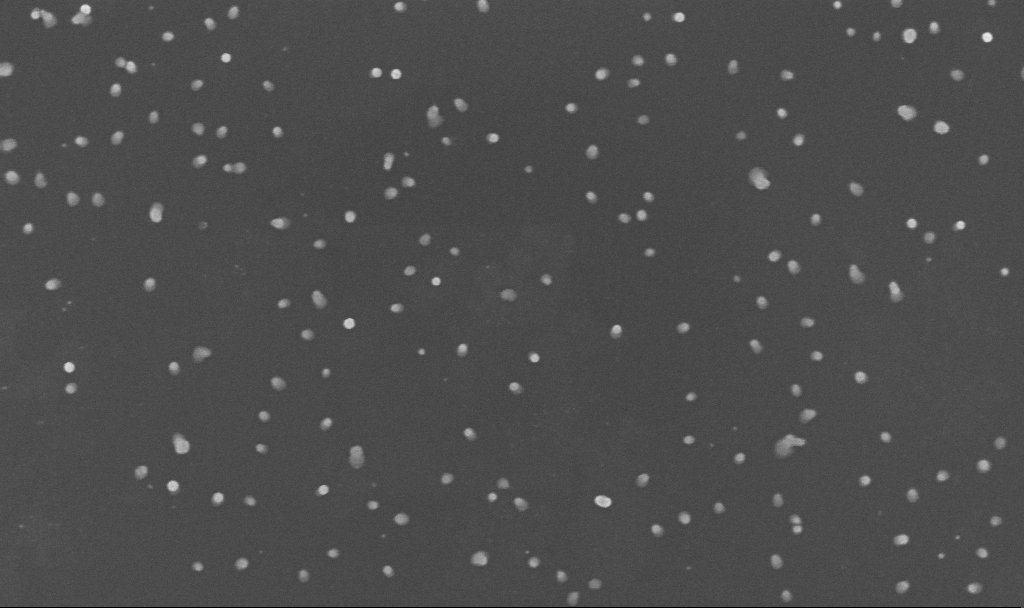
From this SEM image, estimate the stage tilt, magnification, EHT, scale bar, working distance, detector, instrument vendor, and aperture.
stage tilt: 0°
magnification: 150 K X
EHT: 10 kV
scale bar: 100 nm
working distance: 3.4 mm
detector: InLens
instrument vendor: Zeiss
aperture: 30 µm